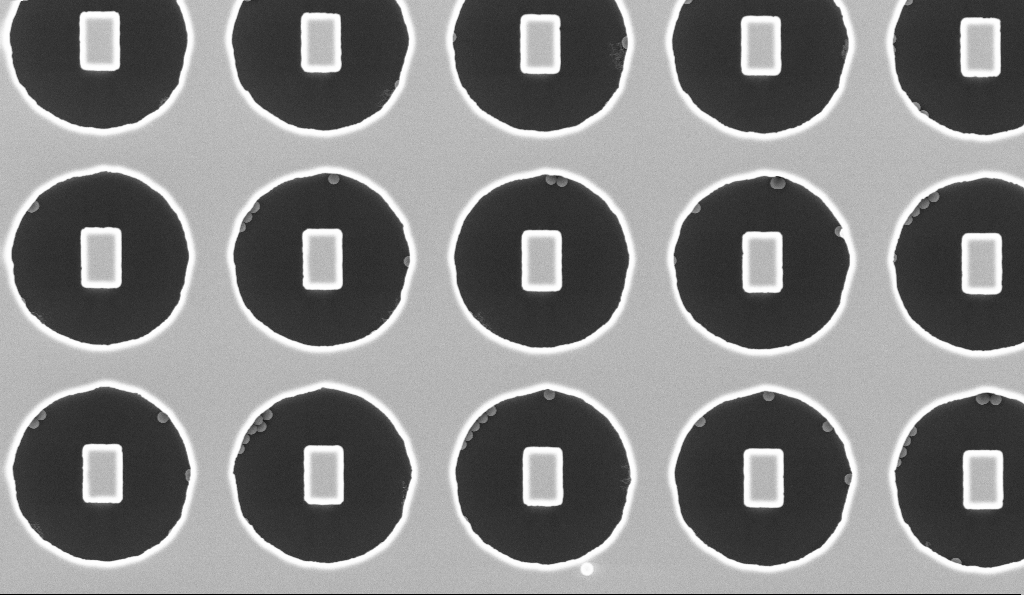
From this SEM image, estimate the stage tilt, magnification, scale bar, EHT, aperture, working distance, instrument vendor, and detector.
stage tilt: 0°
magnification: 7.26 K X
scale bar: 2000 nm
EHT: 5 kV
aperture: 30 µm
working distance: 3.2 mm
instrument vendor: Zeiss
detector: InLens